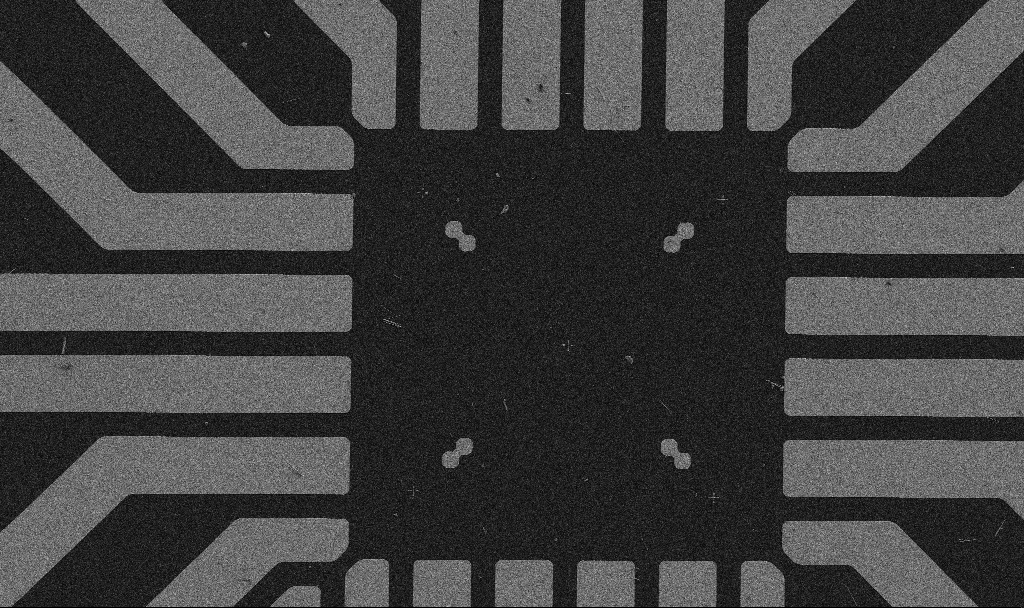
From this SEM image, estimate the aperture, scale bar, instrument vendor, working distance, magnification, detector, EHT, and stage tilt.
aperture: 30 µm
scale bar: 20000 nm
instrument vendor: Zeiss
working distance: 10.7 mm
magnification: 1 K X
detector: SE2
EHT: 5 kV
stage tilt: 0°